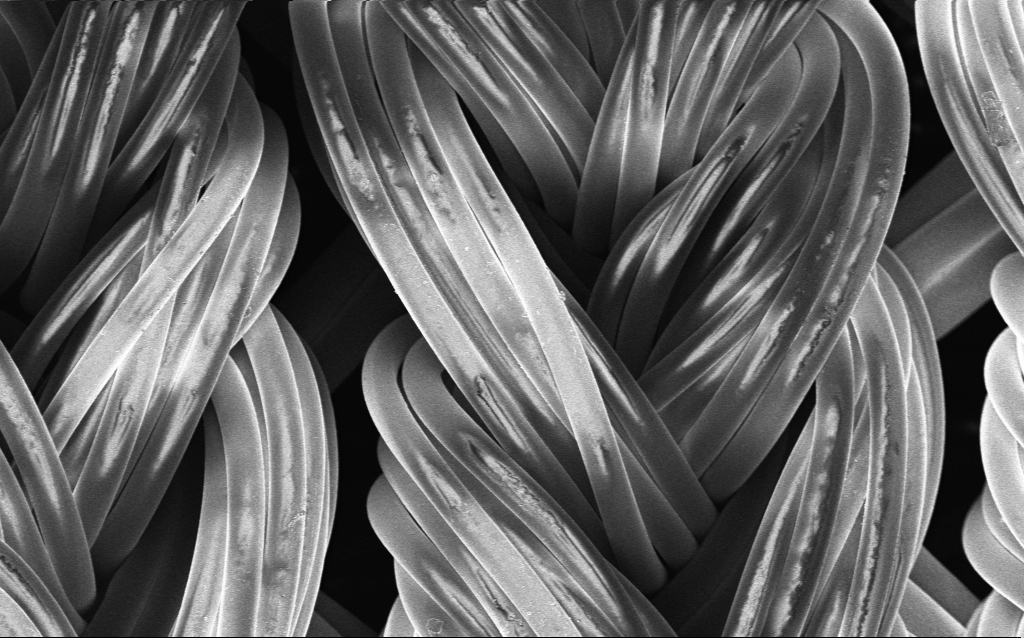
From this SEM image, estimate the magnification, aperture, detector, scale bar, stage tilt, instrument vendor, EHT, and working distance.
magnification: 0.594 K X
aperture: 30 µm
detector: InLens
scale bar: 100000 nm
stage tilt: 0°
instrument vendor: Zeiss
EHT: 2 kV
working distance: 4 mm